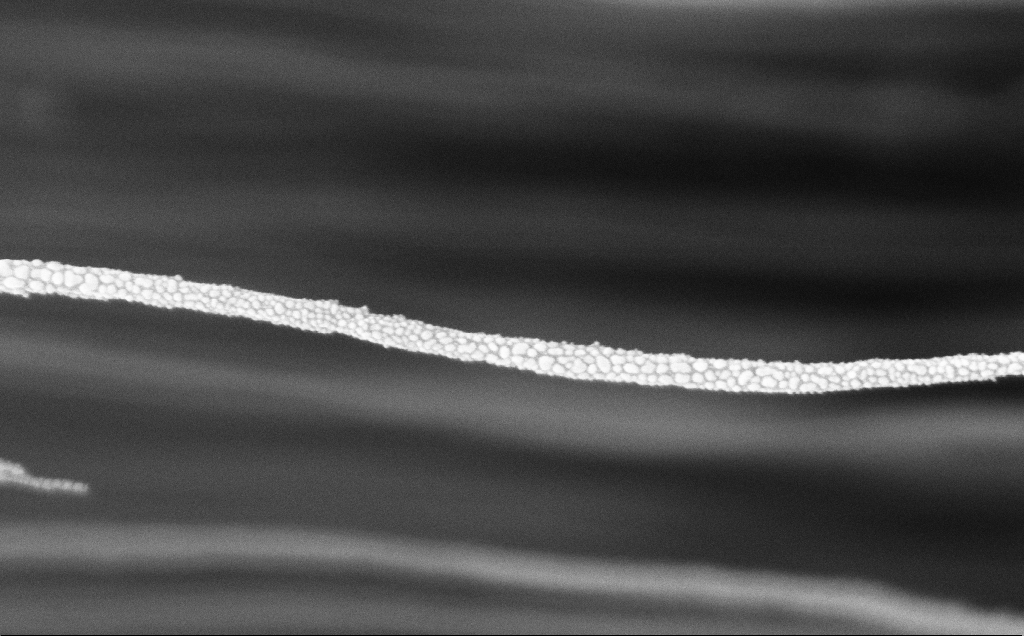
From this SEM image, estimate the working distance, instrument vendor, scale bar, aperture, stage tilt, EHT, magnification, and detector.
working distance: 12 mm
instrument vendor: Zeiss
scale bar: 200 nm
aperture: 30 µm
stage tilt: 0°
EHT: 5 kV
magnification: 89.56 K X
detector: InLens